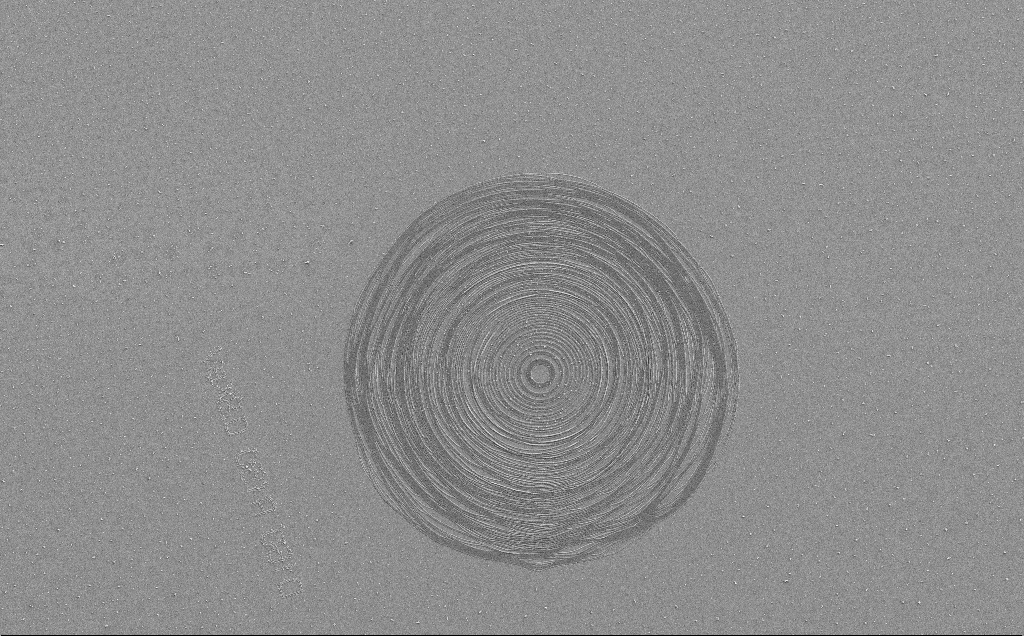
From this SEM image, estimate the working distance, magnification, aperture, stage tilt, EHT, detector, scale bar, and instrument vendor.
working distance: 6 mm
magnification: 0.927 K X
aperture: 30 µm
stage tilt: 0°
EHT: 5 kV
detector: SE2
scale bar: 20000 nm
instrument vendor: Zeiss